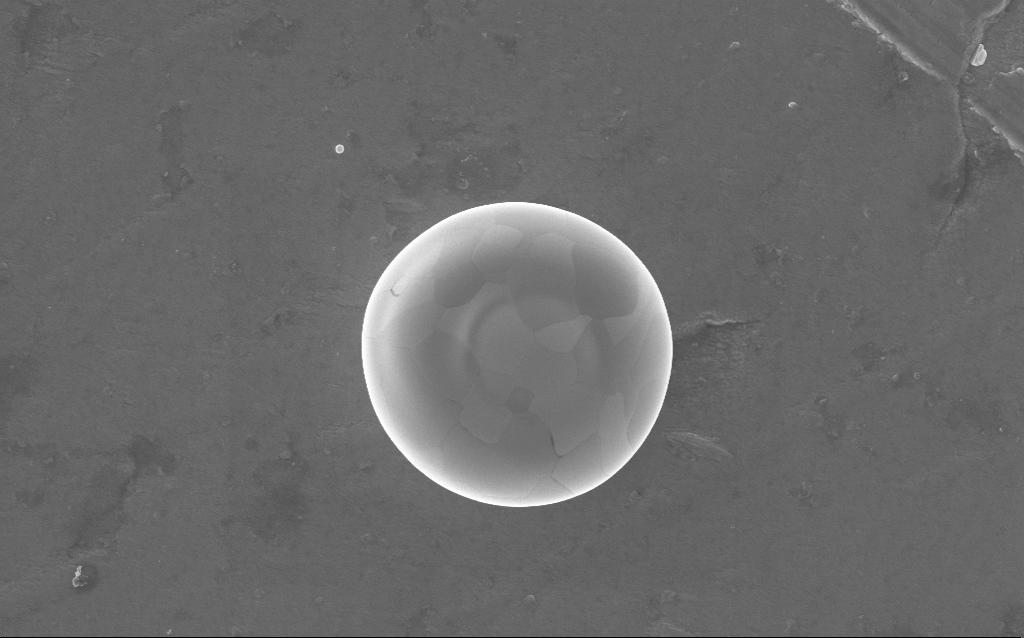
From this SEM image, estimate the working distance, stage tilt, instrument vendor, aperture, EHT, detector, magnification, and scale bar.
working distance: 3 mm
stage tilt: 0°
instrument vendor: Zeiss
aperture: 30 µm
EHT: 5 kV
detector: InLens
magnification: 34 K X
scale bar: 1000 nm